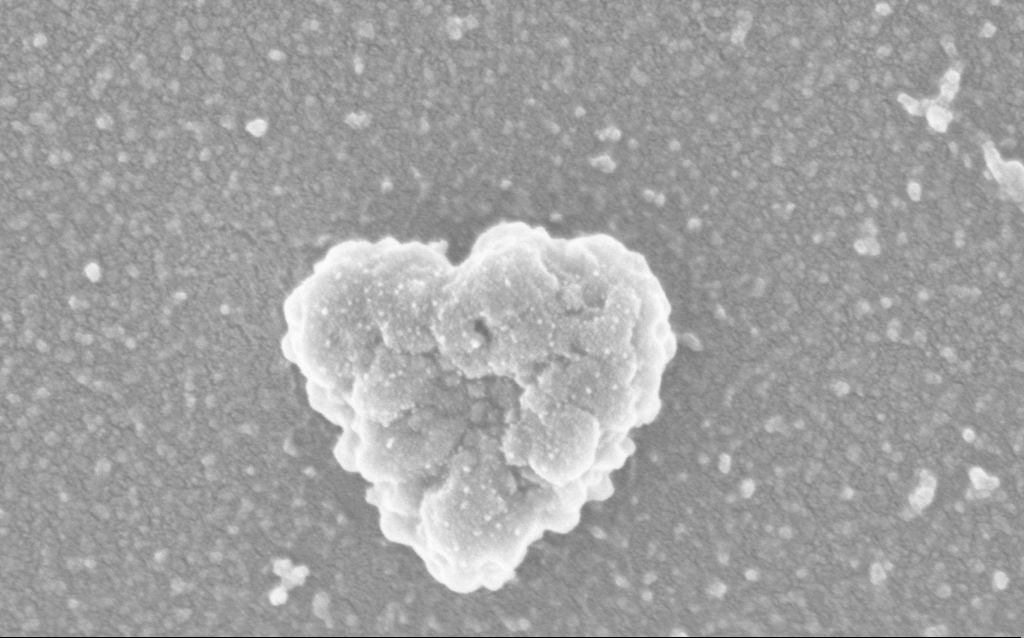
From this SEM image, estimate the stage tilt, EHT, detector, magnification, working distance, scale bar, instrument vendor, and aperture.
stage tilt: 0°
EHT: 3 kV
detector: InLens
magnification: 200 K X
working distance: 2.5 mm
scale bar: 200 nm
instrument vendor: Zeiss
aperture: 30 µm